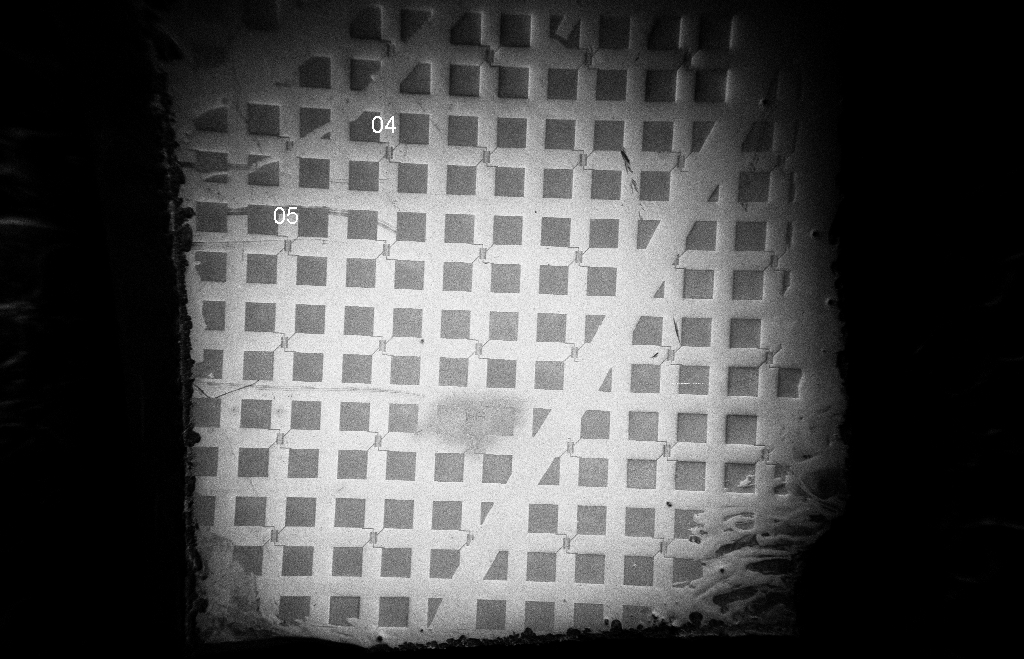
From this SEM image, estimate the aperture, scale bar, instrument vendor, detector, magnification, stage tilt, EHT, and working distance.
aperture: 20 µm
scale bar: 200000 nm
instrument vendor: Zeiss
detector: InLens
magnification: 0.071 K X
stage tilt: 0°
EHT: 5 kV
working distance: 8 mm